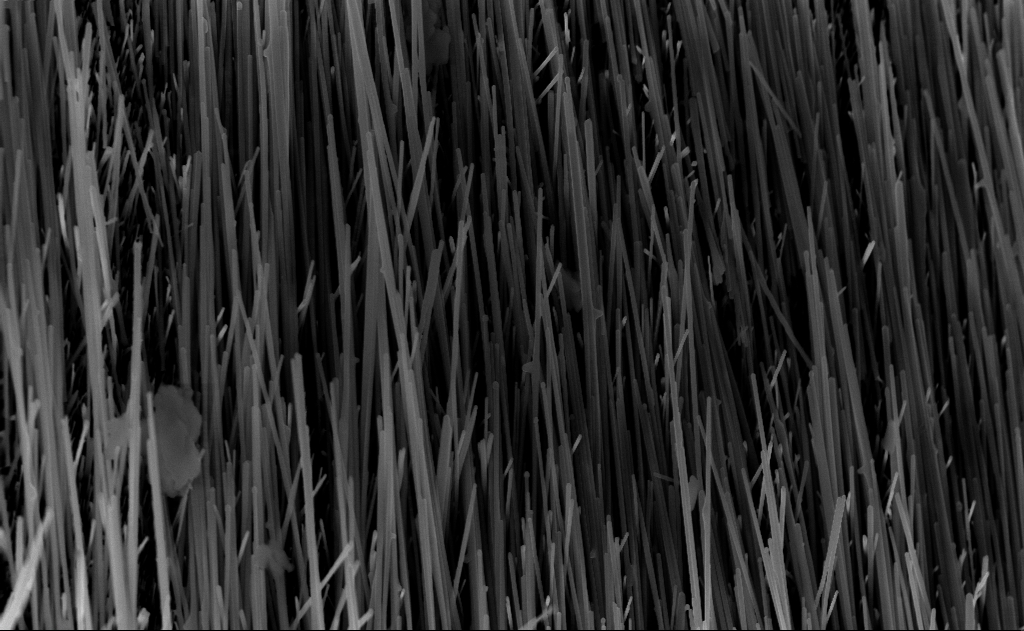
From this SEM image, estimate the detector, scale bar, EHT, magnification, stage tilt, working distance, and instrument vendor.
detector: InLens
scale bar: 1000 nm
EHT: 10 kV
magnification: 20 K X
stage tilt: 0°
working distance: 7 mm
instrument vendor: Zeiss